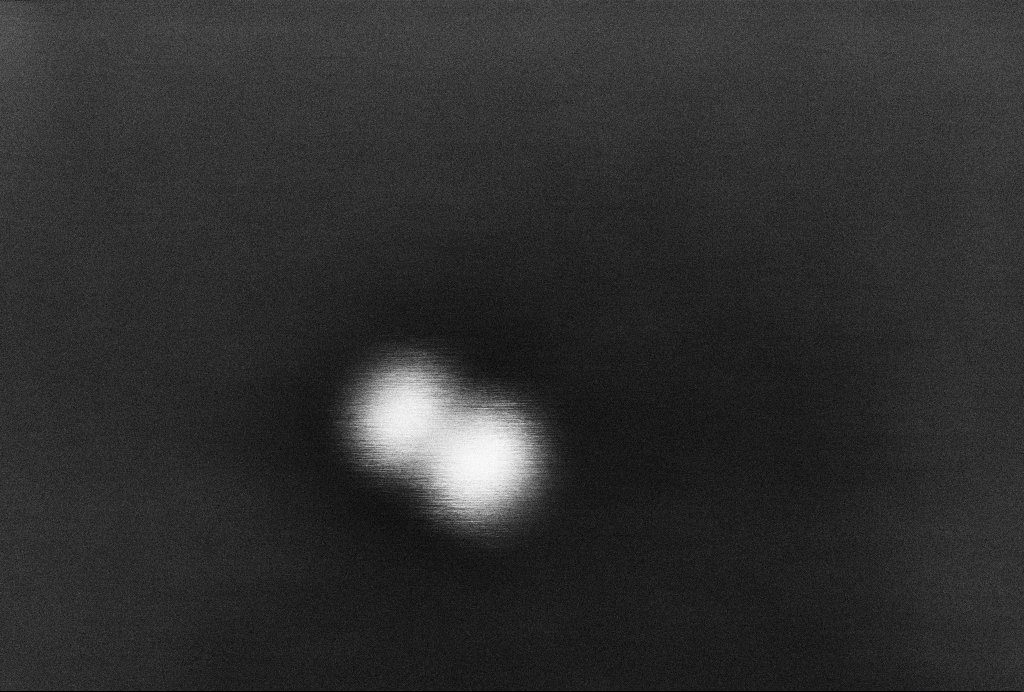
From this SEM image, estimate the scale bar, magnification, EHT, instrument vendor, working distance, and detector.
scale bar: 20 nm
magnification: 1395 K X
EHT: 2 kV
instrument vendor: Zeiss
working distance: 3.3 mm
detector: InLens